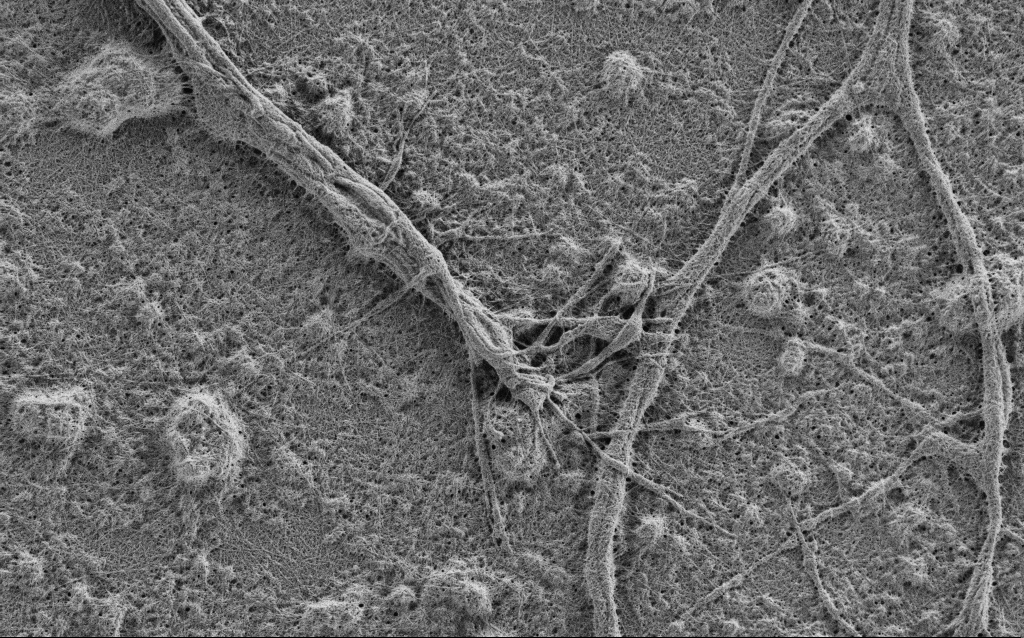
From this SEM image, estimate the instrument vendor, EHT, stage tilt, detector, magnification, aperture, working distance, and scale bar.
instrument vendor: Zeiss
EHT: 0.9 kV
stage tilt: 0°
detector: SE2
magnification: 10 K X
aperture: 30 µm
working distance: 4 mm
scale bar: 2000 nm